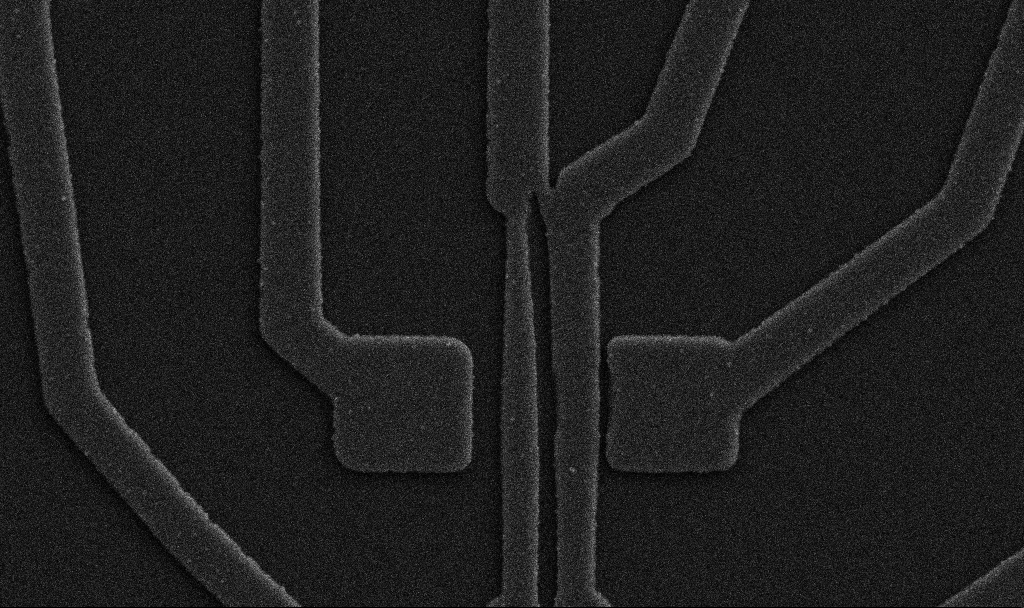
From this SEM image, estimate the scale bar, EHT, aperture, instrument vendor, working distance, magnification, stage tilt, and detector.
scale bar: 2000 nm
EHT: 5 kV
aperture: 30 µm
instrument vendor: Zeiss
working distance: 10.7 mm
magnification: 20 K X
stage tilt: -0°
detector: SE2